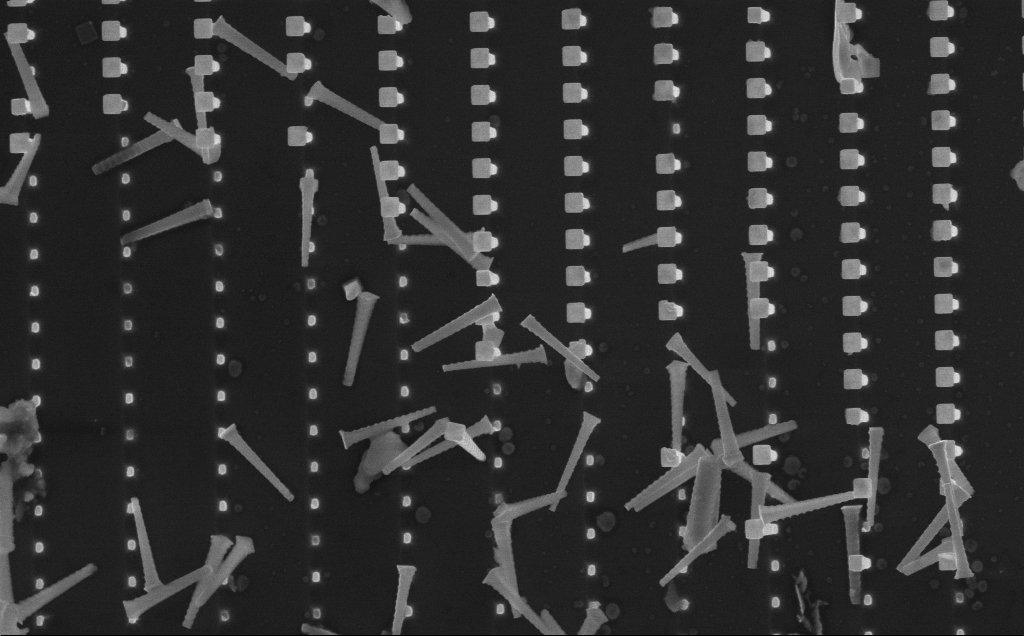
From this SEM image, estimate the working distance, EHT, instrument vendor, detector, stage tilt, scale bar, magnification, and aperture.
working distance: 9 mm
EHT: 10 kV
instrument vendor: Zeiss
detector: InLens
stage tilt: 0°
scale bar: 10000 nm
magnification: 6.8 K X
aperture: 30 µm